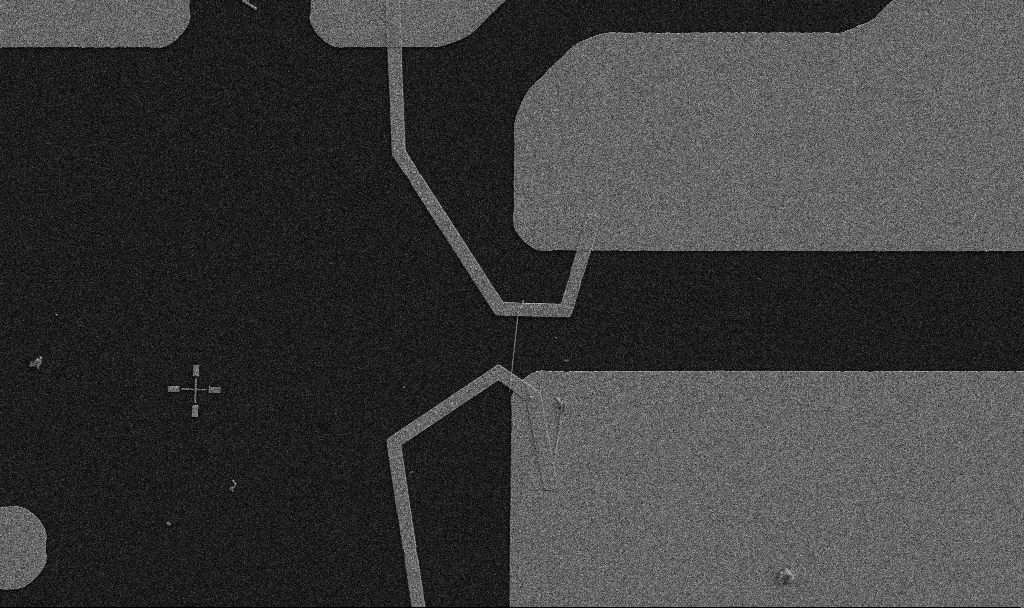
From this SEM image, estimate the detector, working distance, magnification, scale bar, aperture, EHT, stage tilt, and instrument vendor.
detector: SE2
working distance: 10.7 mm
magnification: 5 K X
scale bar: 10000 nm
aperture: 30 µm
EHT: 5 kV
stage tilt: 0°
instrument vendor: Zeiss